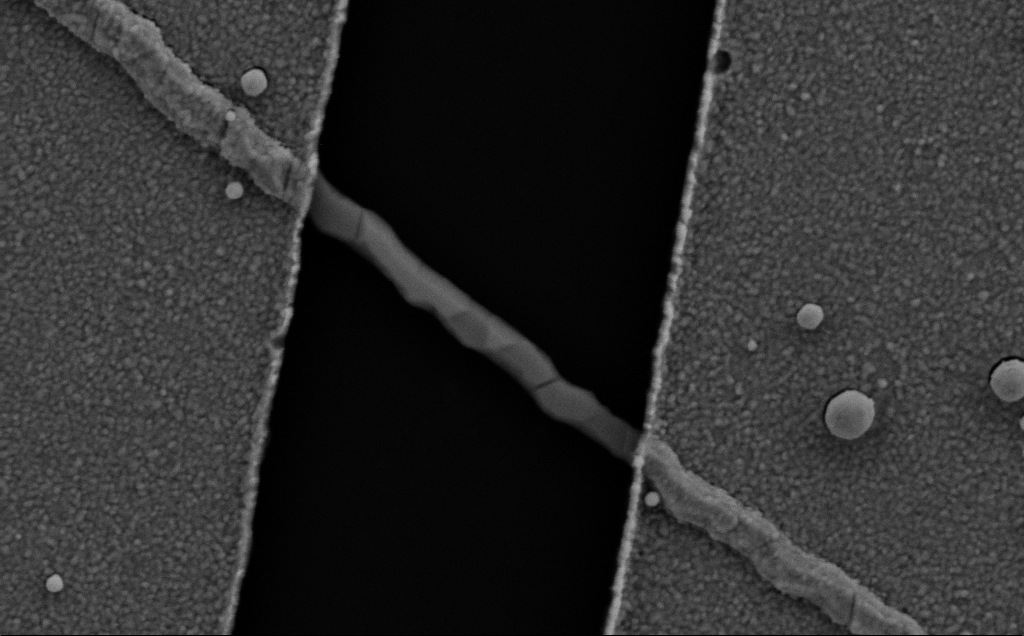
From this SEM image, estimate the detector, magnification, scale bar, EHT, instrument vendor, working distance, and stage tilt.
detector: SE2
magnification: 75.51 K X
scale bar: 200 nm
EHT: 5 kV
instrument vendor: Zeiss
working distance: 8 mm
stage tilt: -0.7°